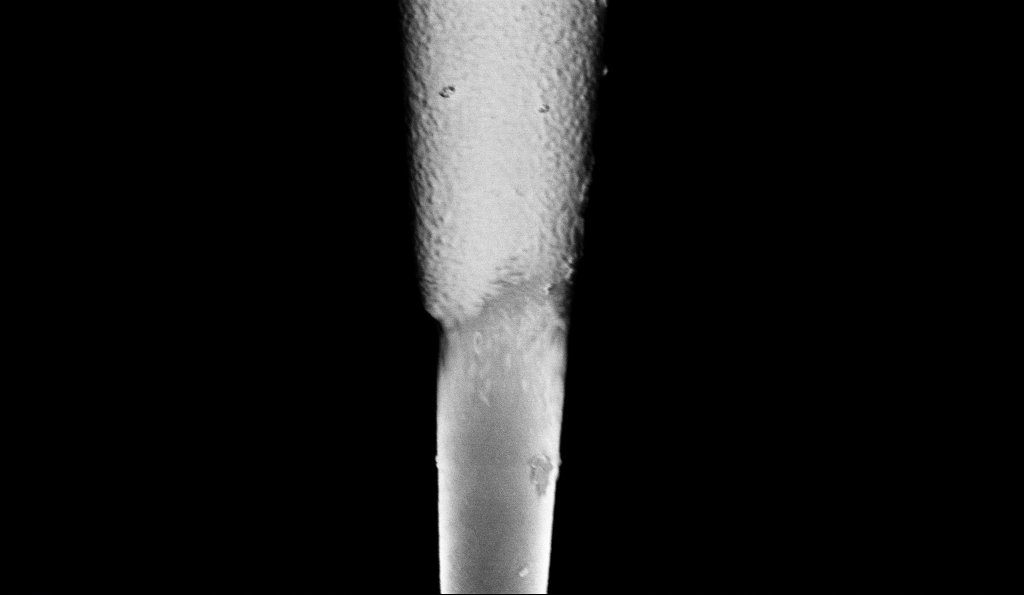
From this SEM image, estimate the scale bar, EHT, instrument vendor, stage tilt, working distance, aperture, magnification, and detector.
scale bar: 2000 nm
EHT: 1 kV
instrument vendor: Zeiss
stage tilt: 0°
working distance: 6.5 mm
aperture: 30 µm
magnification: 25 K X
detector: InLens